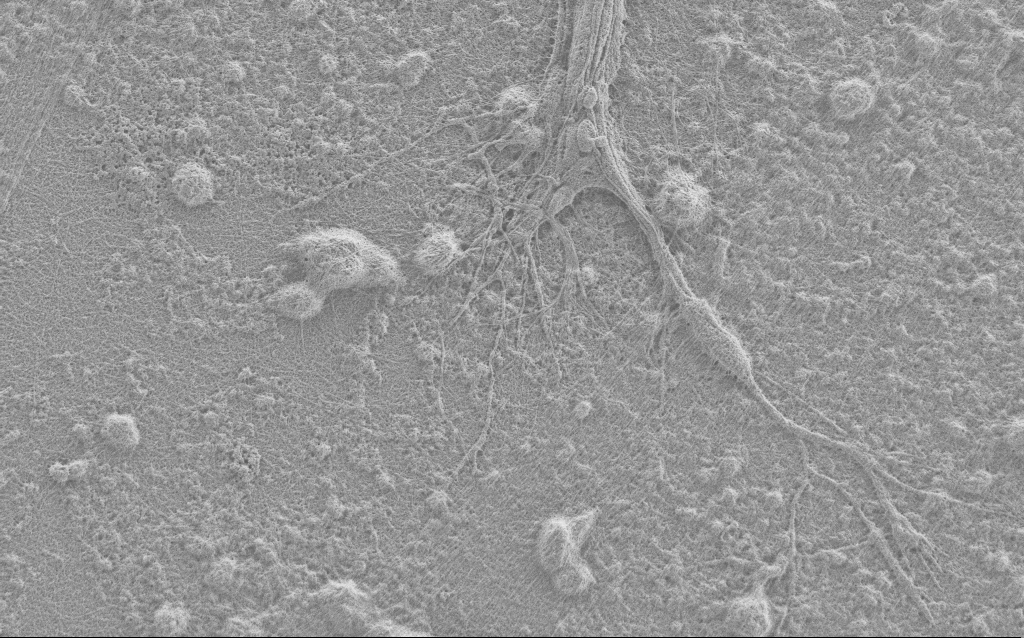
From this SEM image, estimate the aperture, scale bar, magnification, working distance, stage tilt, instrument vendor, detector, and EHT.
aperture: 30 µm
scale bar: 2000 nm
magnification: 7.5 K X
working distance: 4 mm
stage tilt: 0°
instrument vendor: Zeiss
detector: SE2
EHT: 0.9 kV